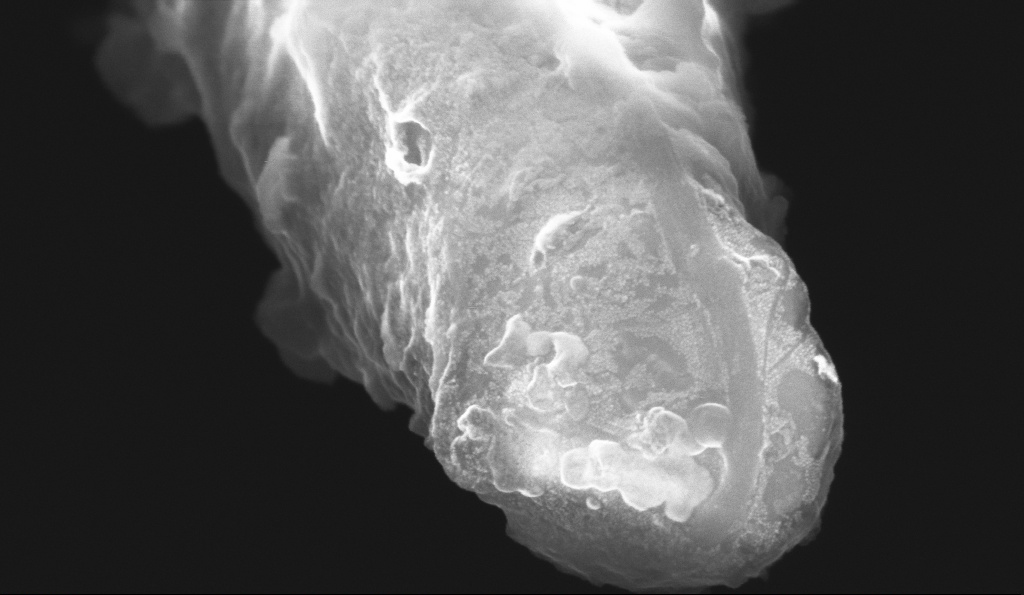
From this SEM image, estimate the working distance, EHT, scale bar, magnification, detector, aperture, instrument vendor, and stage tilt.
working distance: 5.1 mm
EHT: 10 kV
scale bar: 100 nm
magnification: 163.58 K X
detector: InLens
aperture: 30 µm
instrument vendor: Zeiss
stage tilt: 70°